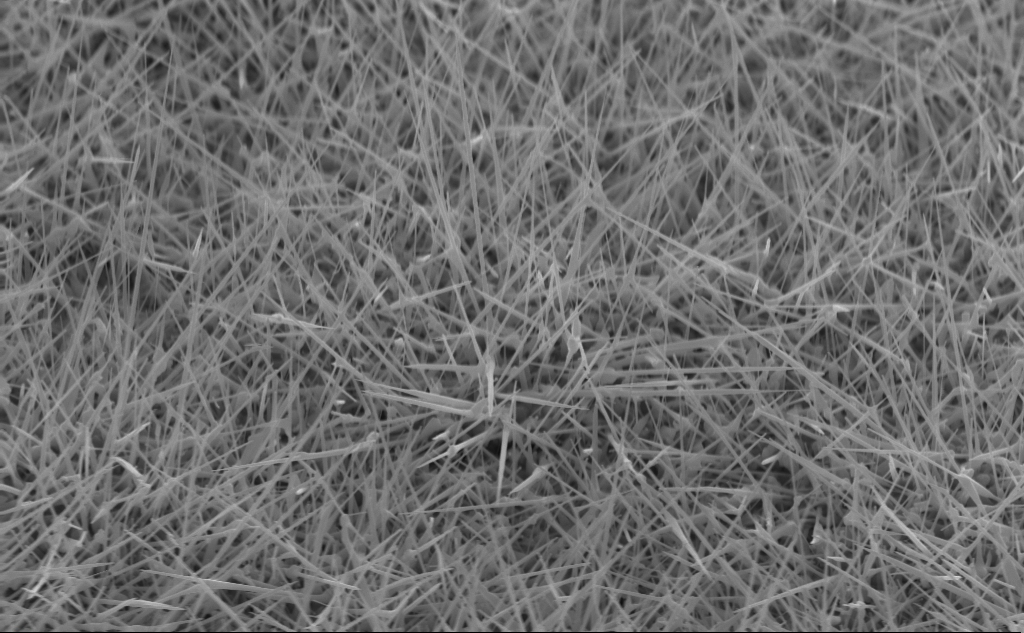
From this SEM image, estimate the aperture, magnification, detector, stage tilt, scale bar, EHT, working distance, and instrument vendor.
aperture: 30 µm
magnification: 20 K X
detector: InLens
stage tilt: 45°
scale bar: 2000 nm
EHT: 10 kV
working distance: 4 mm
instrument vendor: Zeiss